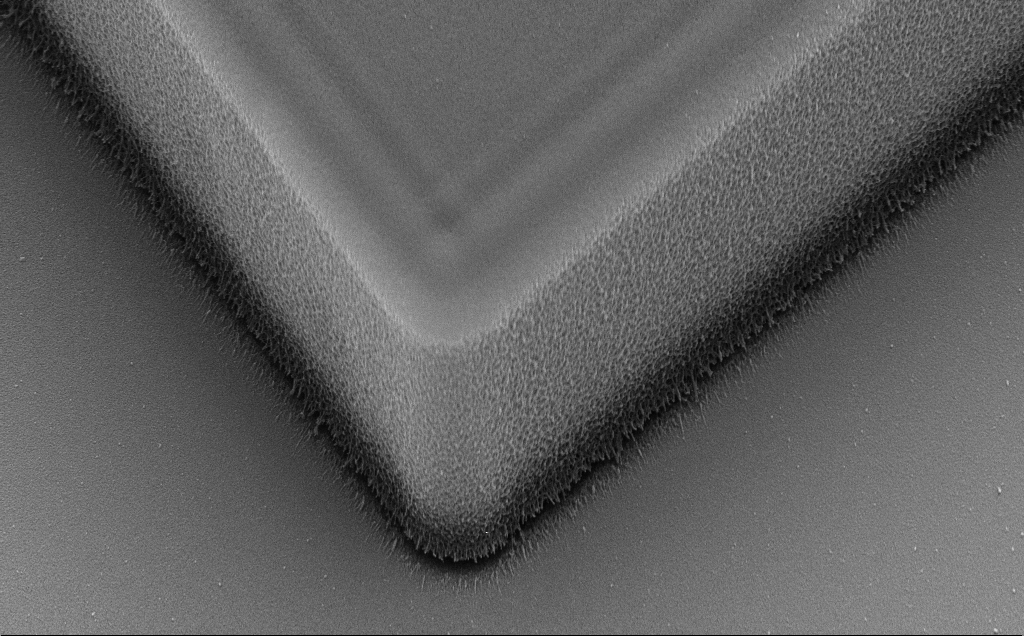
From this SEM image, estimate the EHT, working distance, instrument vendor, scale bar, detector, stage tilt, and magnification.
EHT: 10 kV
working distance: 11 mm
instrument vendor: Zeiss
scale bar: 2000 nm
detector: SE2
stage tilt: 35.3°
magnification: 10.77 K X